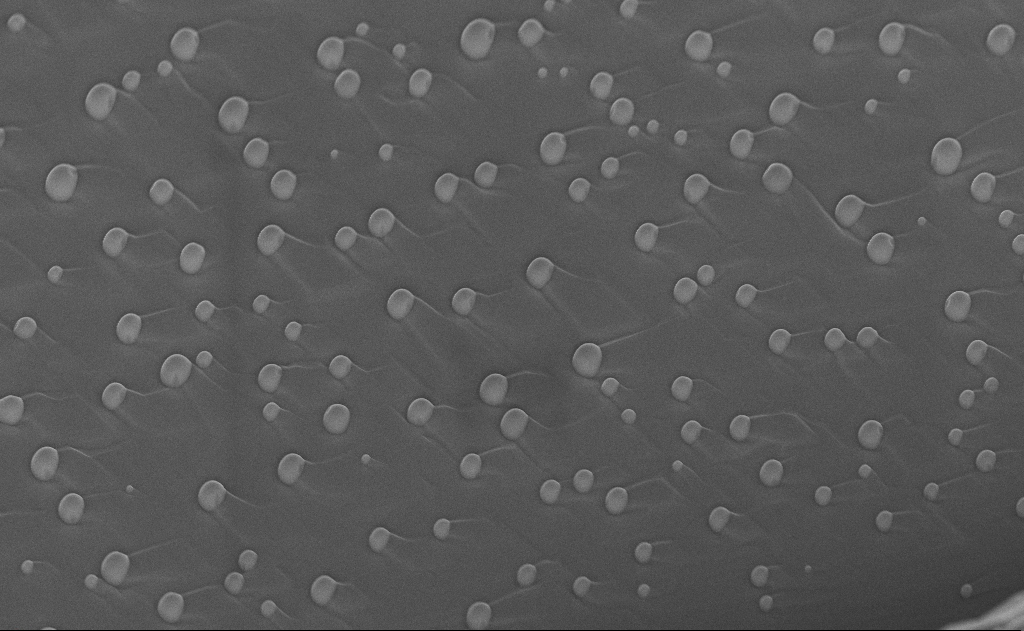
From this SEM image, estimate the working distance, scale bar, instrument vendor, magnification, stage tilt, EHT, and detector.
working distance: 7 mm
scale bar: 2000 nm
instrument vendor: Zeiss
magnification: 9.58 K X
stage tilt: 48.9°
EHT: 10 kV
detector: SE2